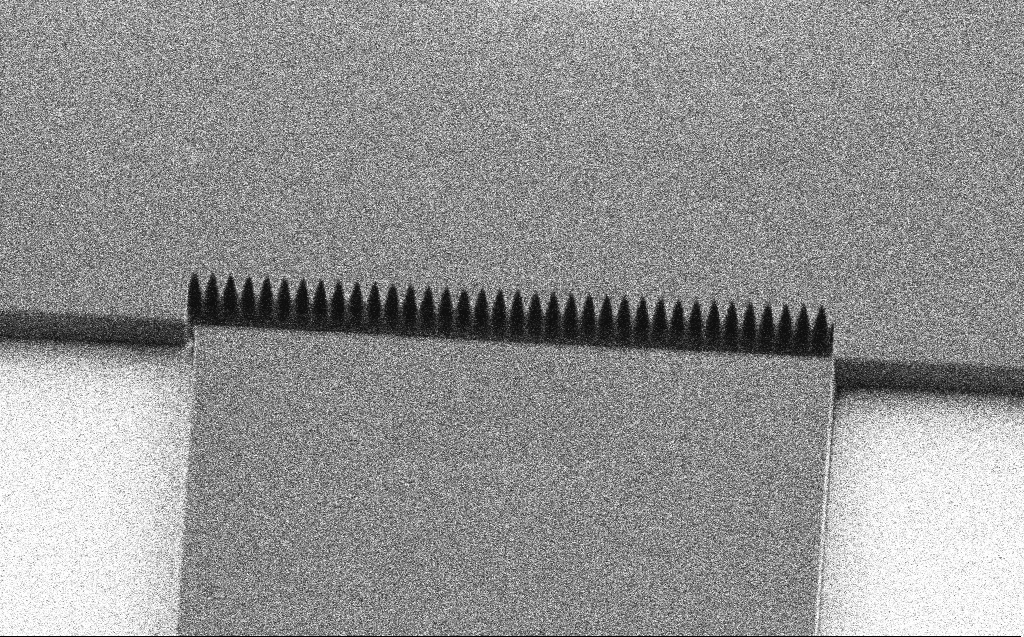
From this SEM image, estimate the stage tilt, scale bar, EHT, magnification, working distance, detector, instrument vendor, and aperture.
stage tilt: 30°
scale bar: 2000 nm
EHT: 1.1 kV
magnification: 7.43 K X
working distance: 6 mm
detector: SE2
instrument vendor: Zeiss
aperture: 30 µm